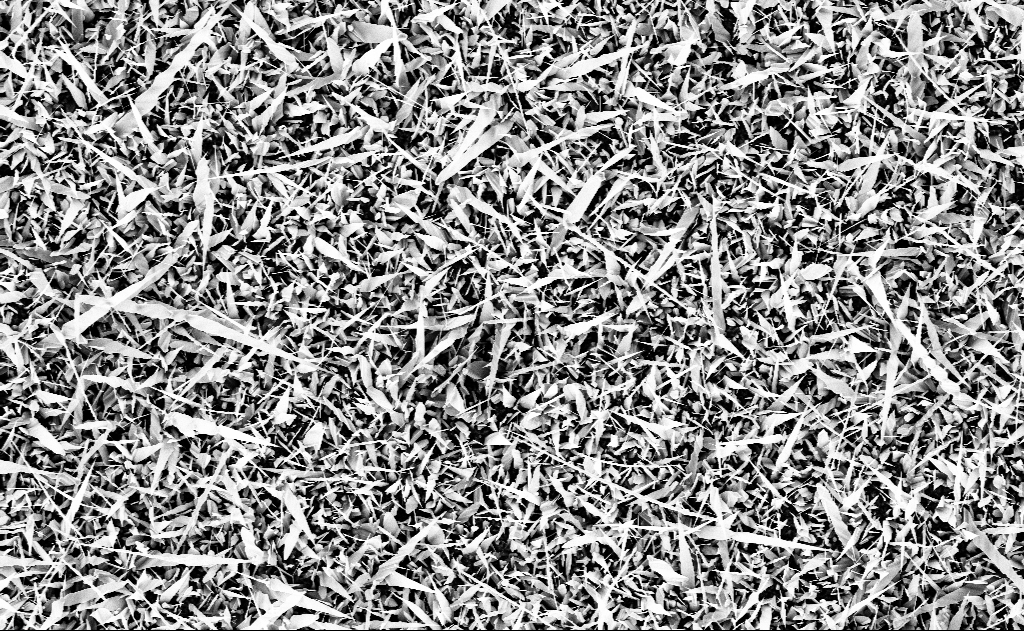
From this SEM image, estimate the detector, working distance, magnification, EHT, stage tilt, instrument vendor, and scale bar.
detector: InLens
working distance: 13 mm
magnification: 5 K X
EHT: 10 kV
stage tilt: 0°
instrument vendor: Zeiss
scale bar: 10000 nm